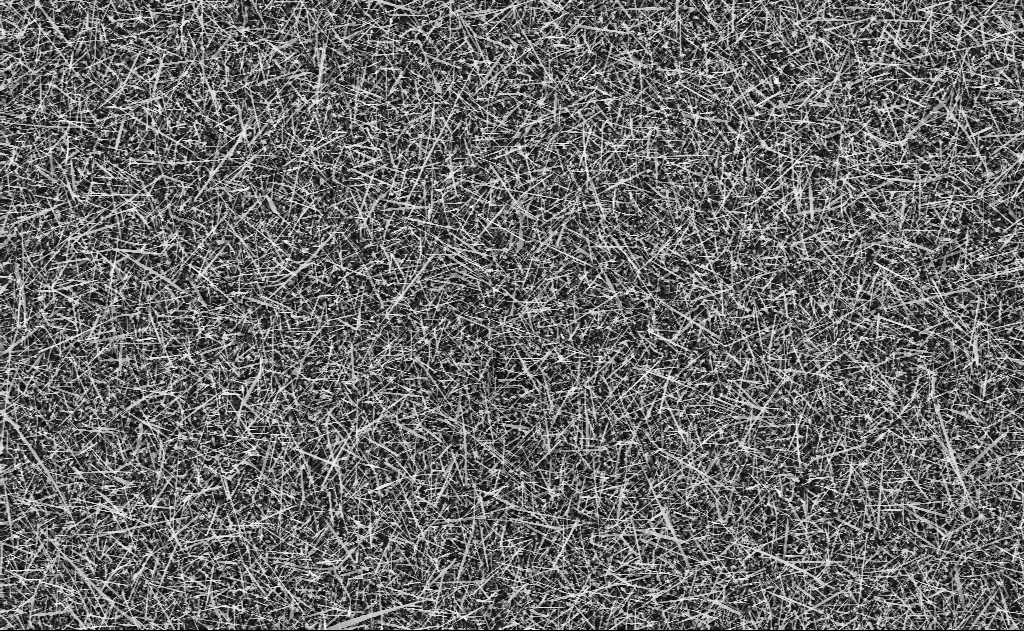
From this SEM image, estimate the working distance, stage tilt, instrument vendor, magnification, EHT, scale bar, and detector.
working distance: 11 mm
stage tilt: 0°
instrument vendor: Zeiss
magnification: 5 K X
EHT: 10 kV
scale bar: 10000 nm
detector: InLens